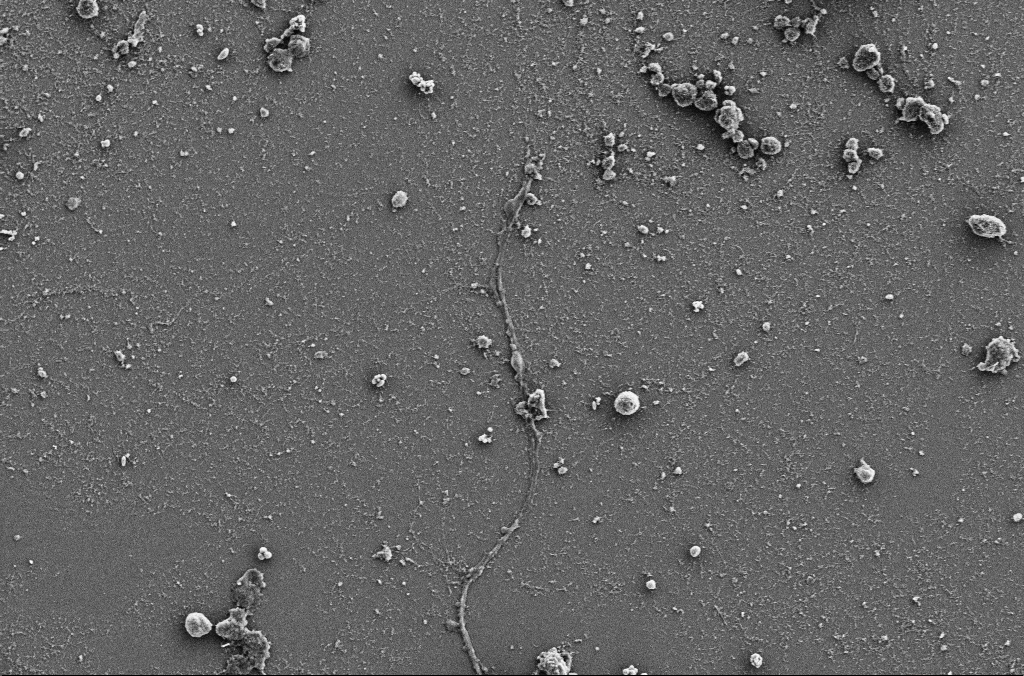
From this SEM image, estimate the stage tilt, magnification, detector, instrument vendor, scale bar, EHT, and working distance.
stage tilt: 0°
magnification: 2.5 K X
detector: SE2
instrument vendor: Zeiss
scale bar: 20000 nm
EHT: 5 kV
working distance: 3.9 mm